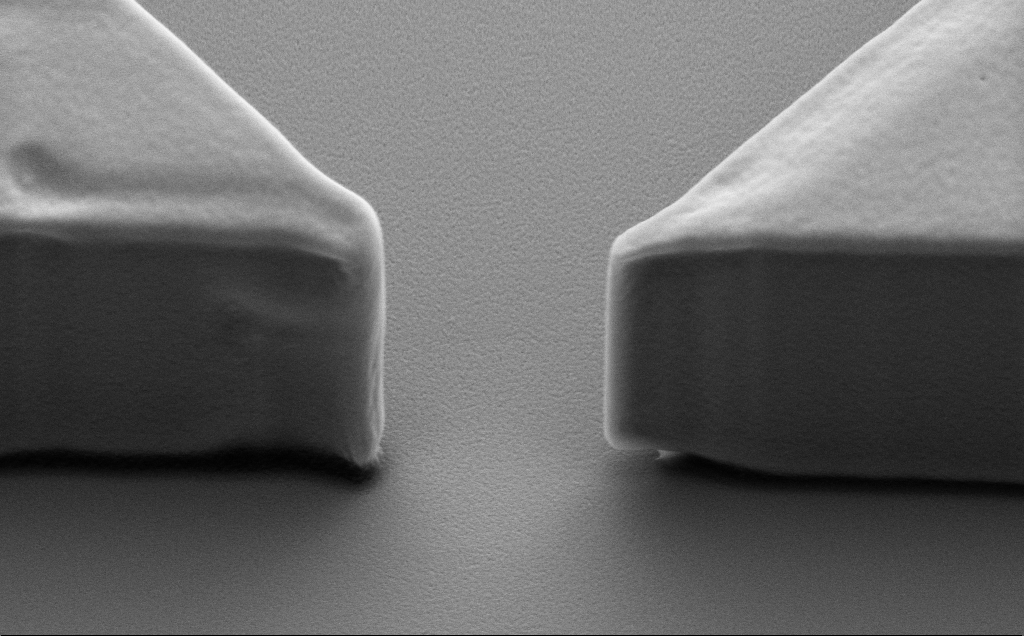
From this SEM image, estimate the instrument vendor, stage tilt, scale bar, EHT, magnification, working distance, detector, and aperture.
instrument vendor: Zeiss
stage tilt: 40°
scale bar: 1000 nm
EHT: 5 kV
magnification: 15 K X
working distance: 9 mm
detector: SE2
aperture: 30 µm